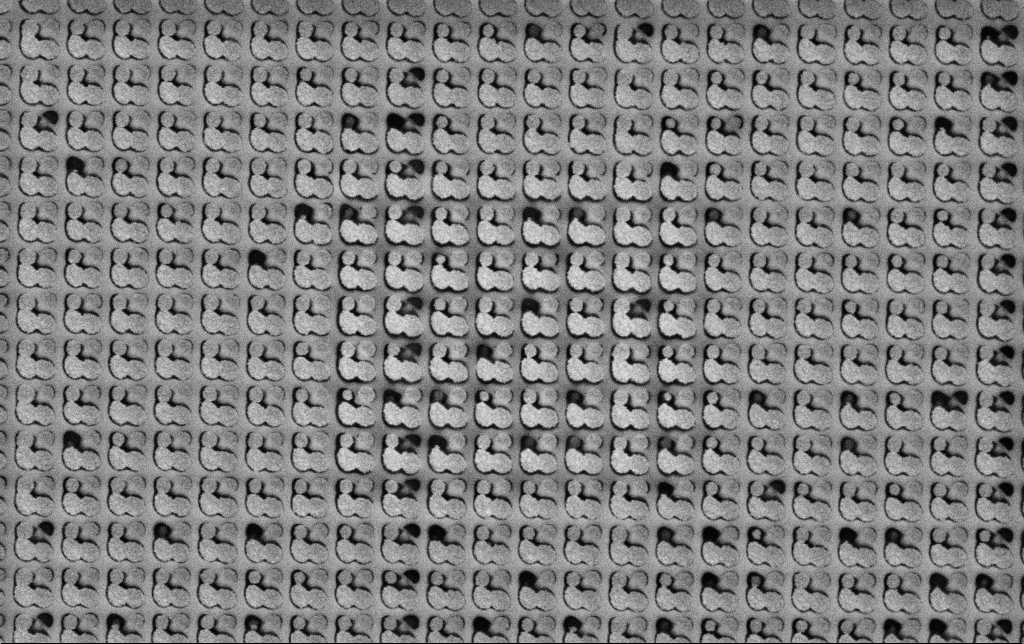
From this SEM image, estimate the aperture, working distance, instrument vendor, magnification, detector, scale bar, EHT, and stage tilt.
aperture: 30 µm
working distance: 6.1 mm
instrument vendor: Zeiss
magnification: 36.35 K X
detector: SE2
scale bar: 1000 nm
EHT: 3 kV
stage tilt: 0°